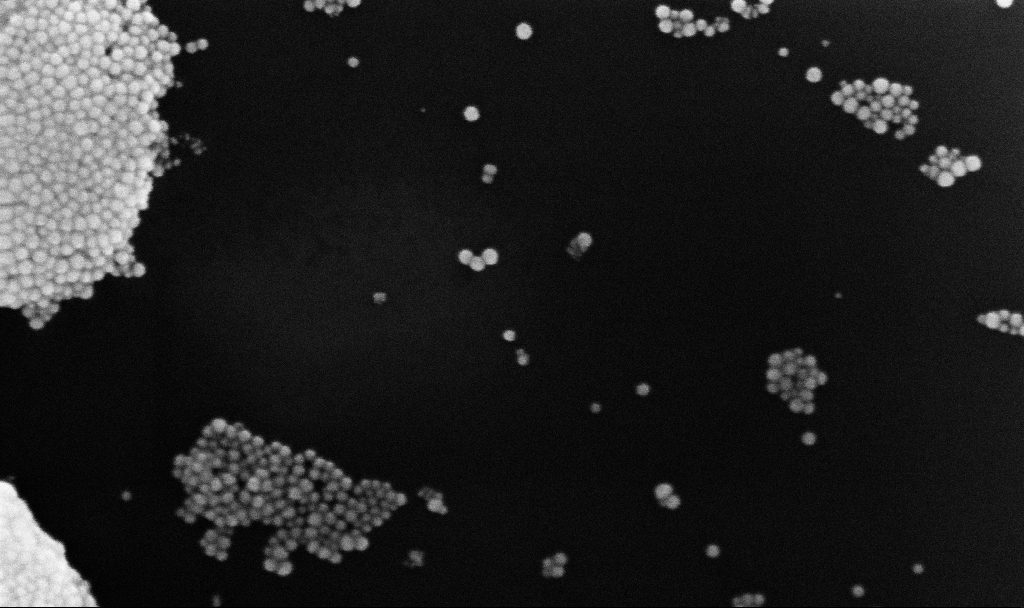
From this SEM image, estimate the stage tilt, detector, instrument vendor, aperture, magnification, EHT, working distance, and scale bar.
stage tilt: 0°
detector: InLens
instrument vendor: Zeiss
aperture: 30 µm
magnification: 271.23 K X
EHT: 10 kV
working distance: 3.1 mm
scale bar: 100 nm